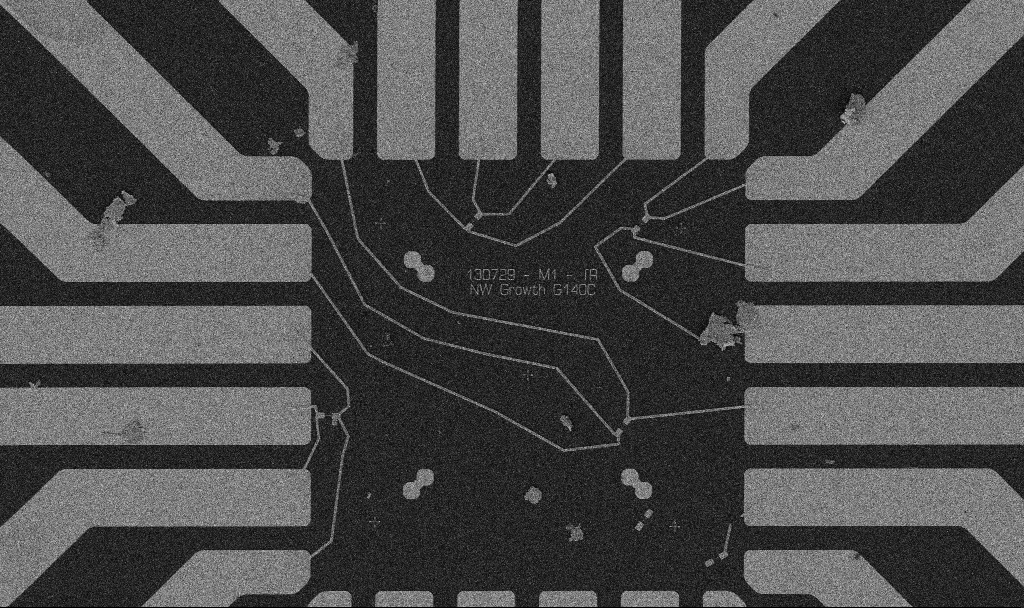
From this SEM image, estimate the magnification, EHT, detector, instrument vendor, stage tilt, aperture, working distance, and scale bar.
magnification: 1 K X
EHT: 5 kV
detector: SE2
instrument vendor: Zeiss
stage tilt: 0°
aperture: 30 µm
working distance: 10.7 mm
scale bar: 20000 nm